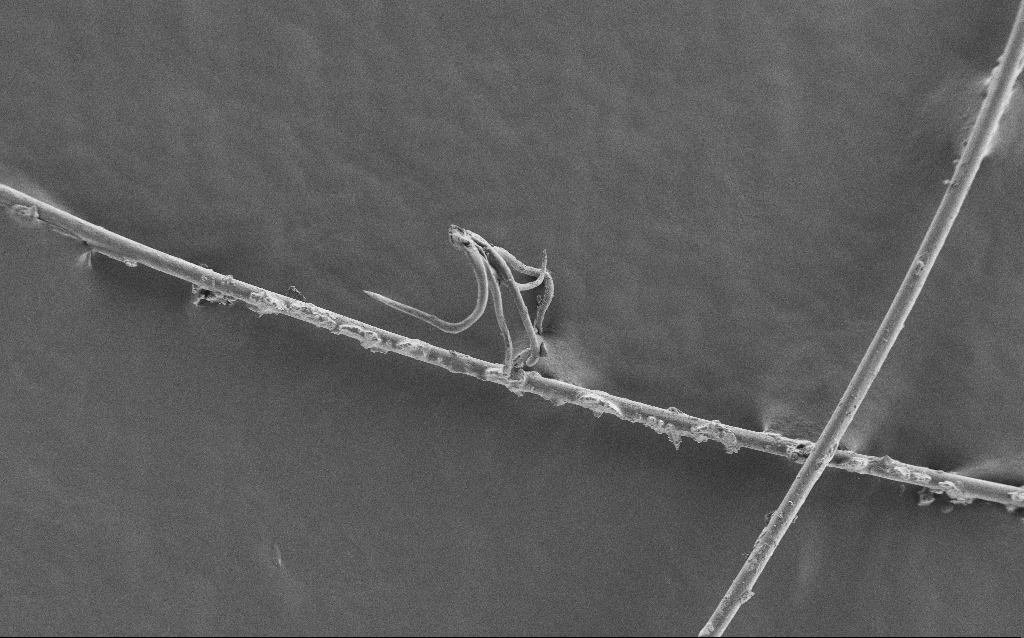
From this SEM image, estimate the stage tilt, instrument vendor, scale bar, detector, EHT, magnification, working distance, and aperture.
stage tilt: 0°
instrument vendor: Zeiss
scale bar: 100000 nm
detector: SE2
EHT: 1 kV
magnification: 0.292 K X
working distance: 5 mm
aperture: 30 µm